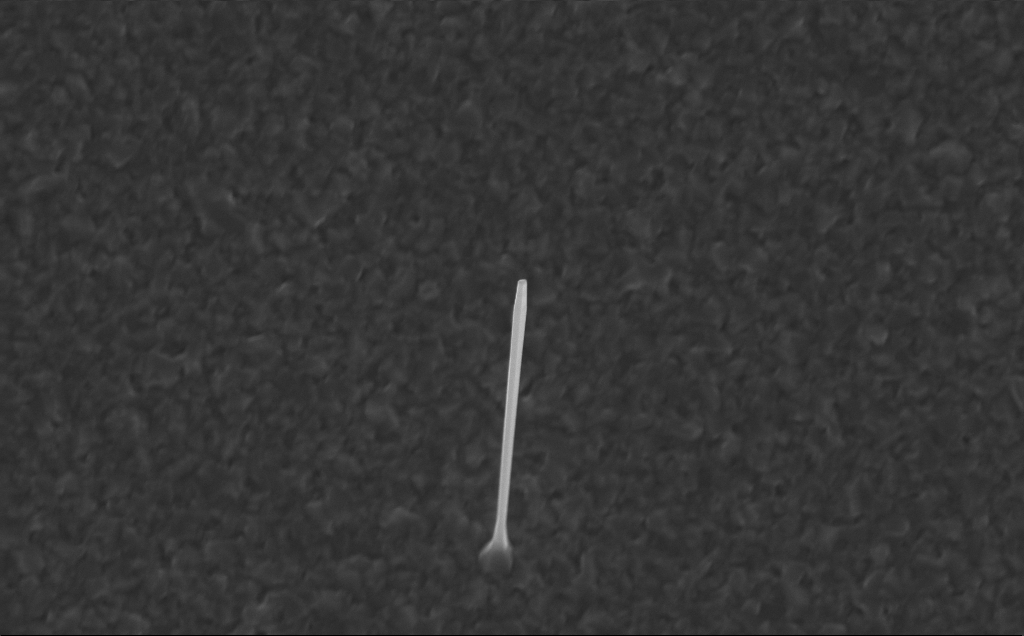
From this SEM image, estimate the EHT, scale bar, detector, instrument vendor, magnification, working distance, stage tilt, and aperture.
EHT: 10 kV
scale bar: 2000 nm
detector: InLens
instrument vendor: Zeiss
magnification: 20 K X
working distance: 5 mm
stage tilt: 0°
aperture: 30 µm